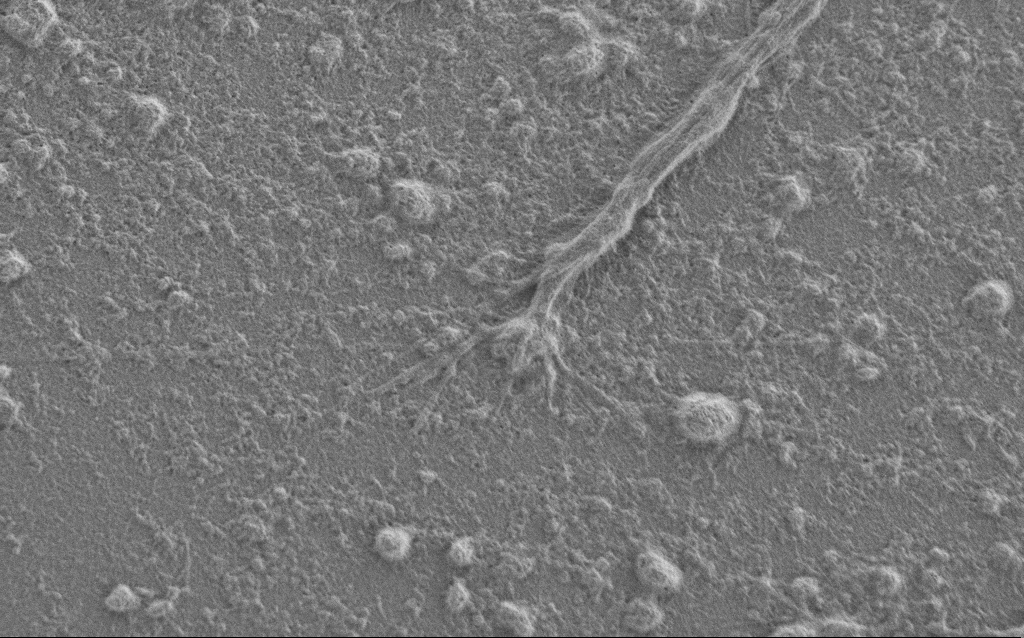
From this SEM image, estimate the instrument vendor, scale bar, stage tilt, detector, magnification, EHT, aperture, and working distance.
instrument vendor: Zeiss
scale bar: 2000 nm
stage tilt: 0°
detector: SE2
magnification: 7.5 K X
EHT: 1 kV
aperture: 30 µm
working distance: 6 mm